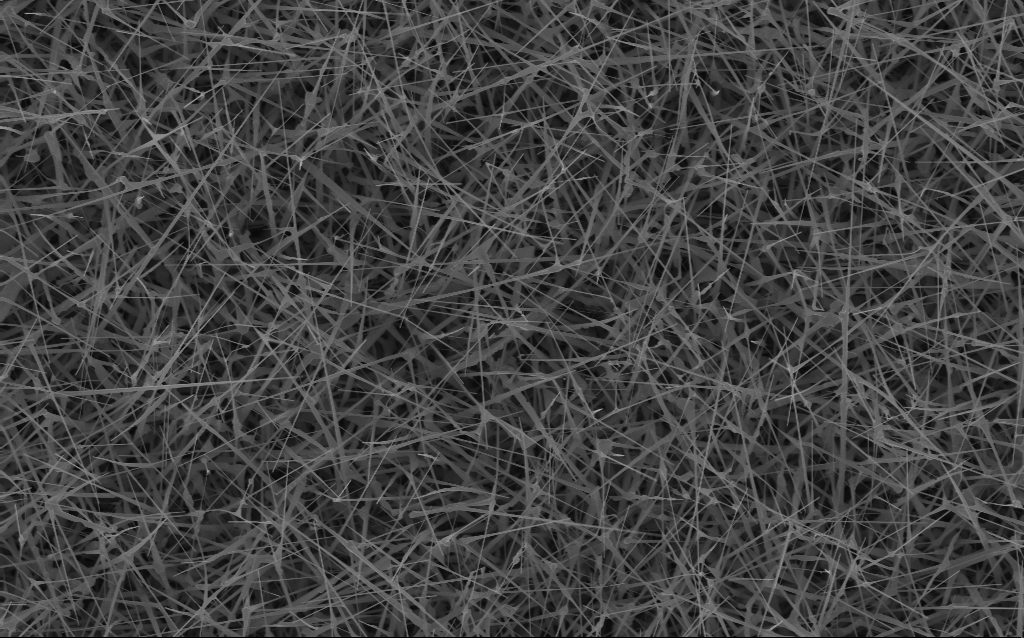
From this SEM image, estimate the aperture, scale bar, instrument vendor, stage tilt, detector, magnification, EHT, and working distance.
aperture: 30 µm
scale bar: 2000 nm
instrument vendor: Zeiss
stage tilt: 0°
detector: InLens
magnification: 10 K X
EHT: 10 kV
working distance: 6 mm